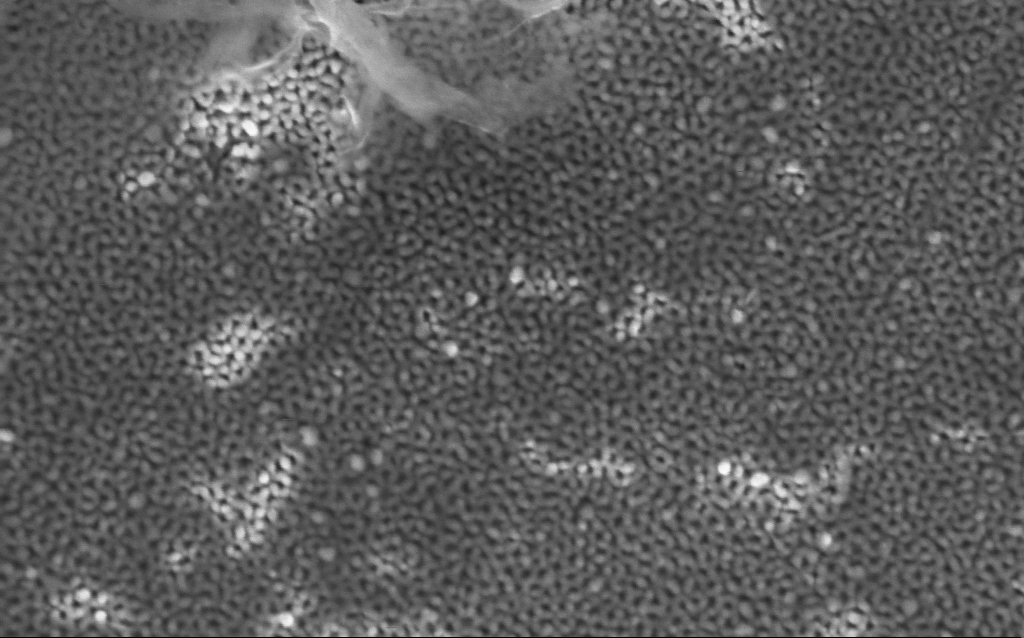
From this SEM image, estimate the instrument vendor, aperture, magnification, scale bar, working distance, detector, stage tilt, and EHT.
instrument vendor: Zeiss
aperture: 30 µm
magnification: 160.72 K X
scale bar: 100 nm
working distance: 4.1 mm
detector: InLens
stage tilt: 0.1°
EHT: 5 kV